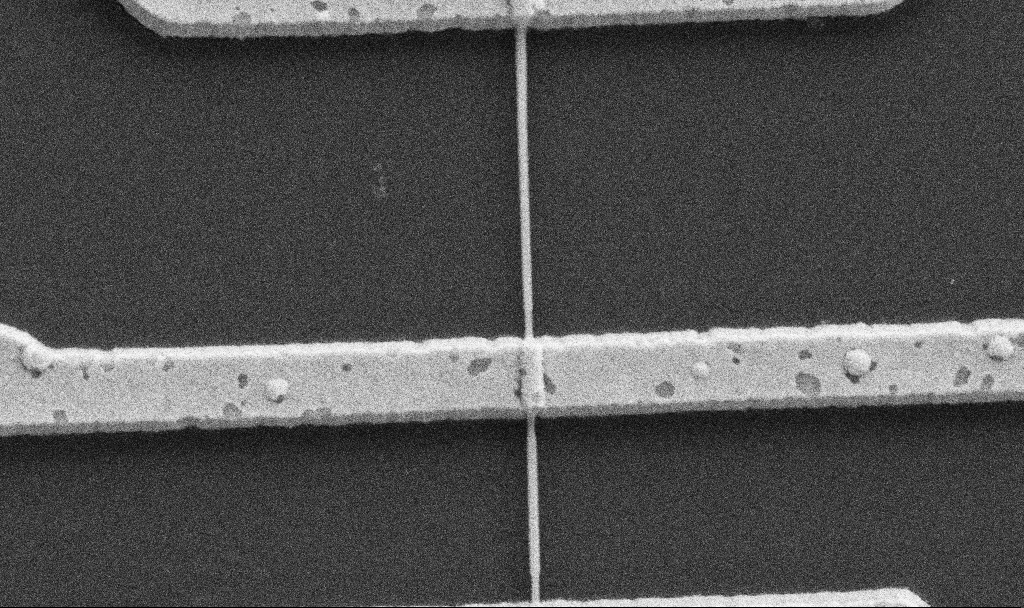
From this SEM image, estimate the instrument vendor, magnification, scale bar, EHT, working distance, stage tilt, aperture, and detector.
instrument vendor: Zeiss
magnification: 51.16 K X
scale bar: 1000 nm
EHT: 5 kV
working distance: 10.6 mm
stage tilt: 0°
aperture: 30 µm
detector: SE2